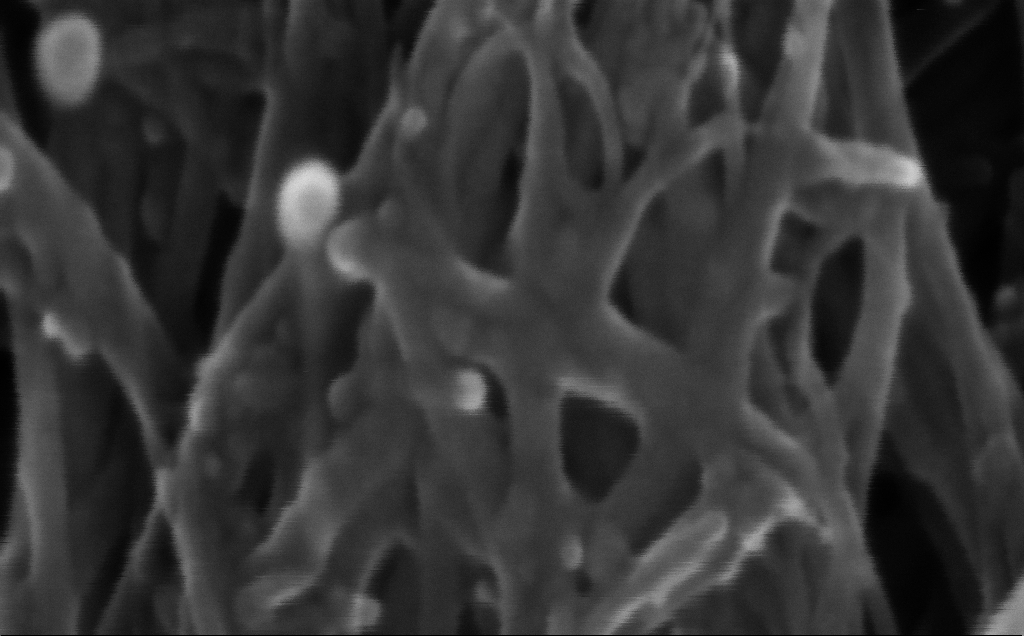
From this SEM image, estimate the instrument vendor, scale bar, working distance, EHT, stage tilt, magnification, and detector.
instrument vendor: Zeiss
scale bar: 20 nm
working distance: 4 mm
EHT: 5 kV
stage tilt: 0°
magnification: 866.06 K X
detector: InLens